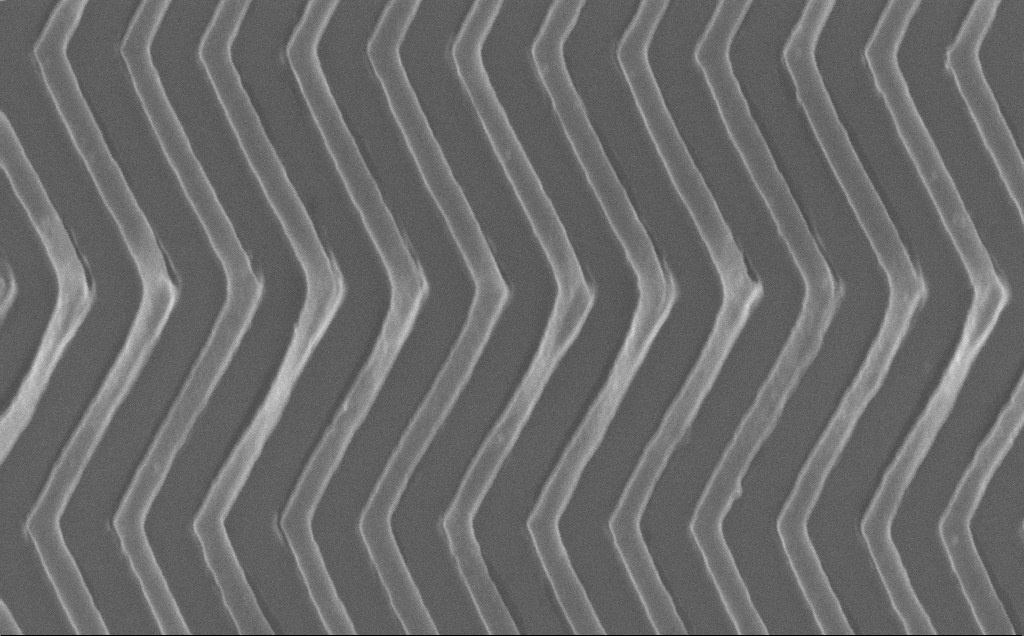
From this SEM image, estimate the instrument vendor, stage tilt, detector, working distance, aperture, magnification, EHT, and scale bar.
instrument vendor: Zeiss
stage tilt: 0°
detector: InLens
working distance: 7 mm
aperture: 30 µm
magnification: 87.18 K X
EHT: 10 kV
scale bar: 200 nm